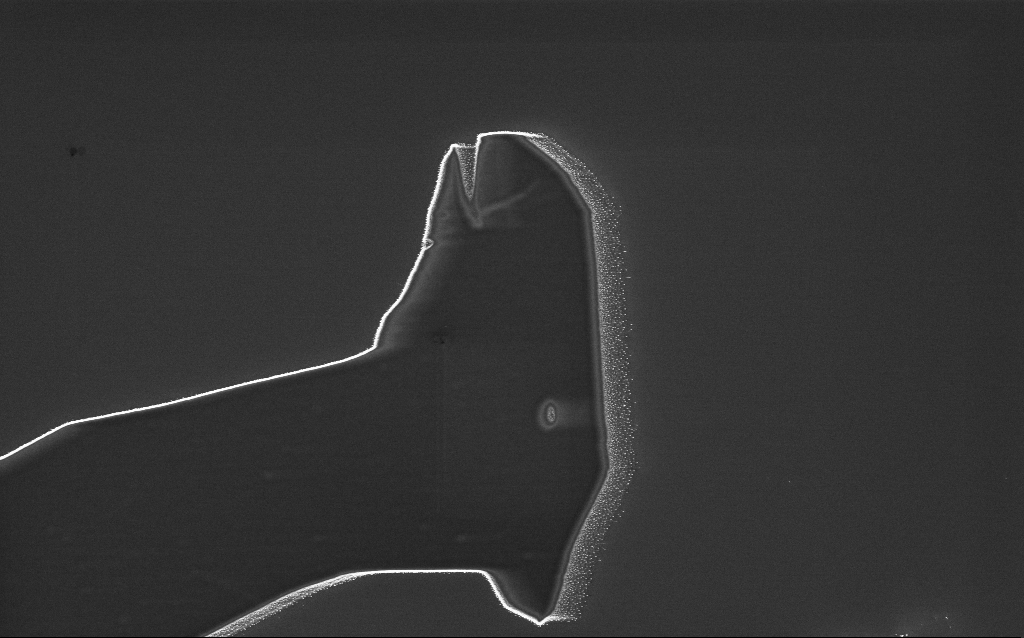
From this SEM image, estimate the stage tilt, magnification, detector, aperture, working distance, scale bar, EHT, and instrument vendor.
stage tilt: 0°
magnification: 2.93 K X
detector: InLens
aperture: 30 µm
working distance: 5 mm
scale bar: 10000 nm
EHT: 3 kV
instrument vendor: Zeiss